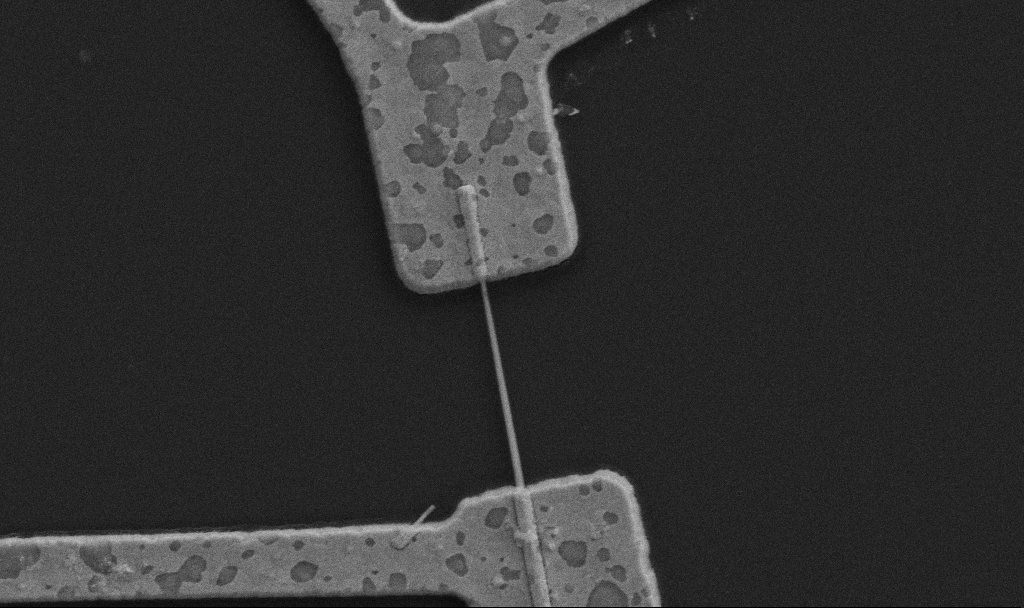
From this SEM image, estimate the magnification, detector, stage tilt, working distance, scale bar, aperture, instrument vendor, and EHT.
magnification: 30 K X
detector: SE2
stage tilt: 0°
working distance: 10.7 mm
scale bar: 1000 nm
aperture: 30 µm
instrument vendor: Zeiss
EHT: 5 kV